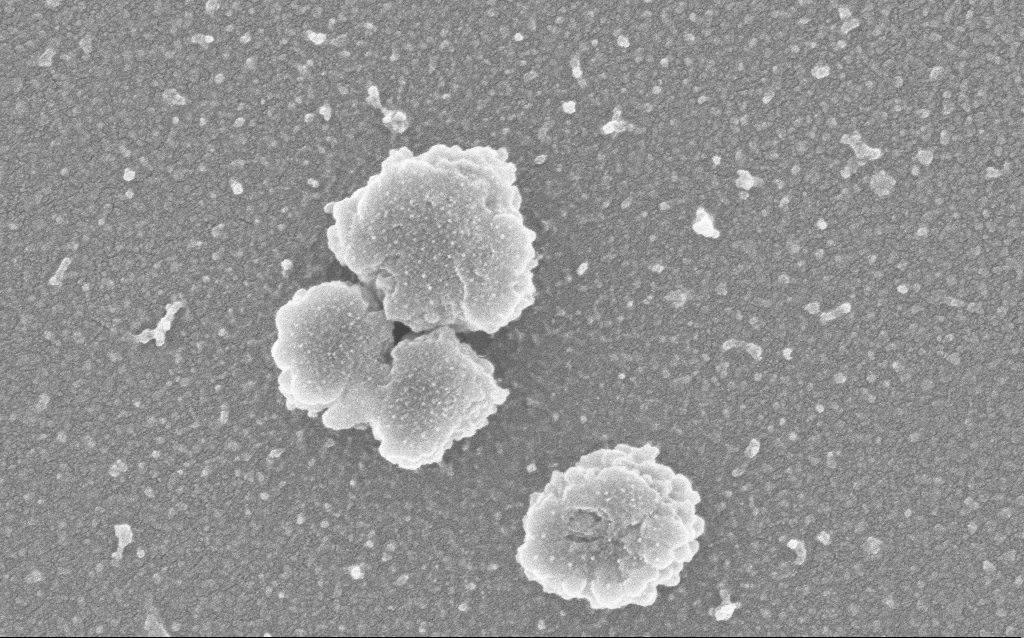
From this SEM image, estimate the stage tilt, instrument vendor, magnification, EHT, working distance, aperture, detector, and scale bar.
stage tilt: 0°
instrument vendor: Zeiss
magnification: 120 K X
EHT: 3 kV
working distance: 2.5 mm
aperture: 30 µm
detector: InLens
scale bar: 200 nm